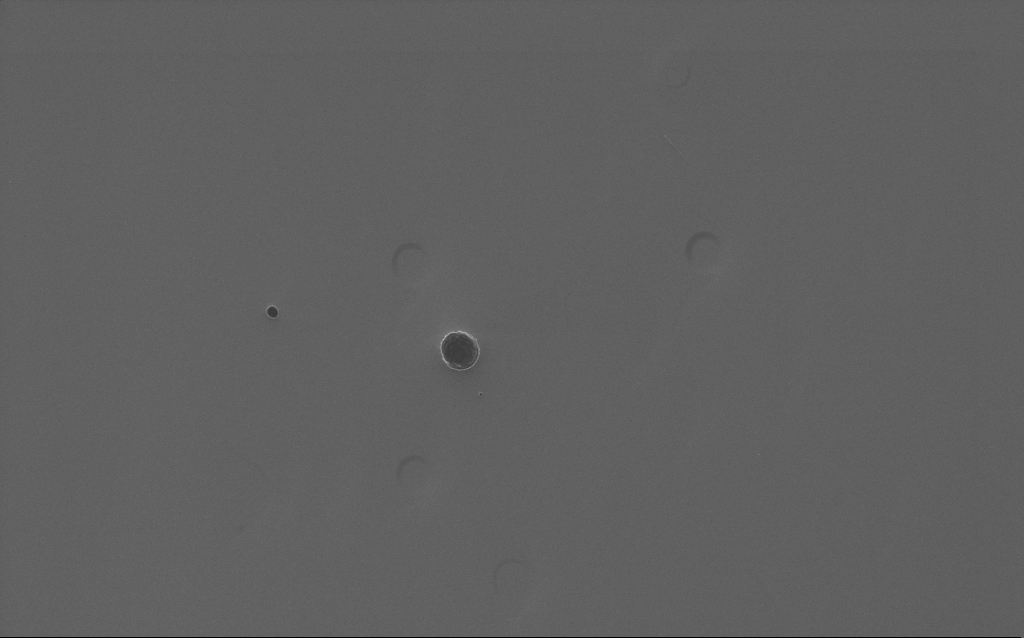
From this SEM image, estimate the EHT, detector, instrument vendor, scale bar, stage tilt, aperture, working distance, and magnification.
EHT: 5 kV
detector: InLens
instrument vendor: Zeiss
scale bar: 2000 nm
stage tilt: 0°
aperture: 30 µm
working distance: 4 mm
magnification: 9.2 K X